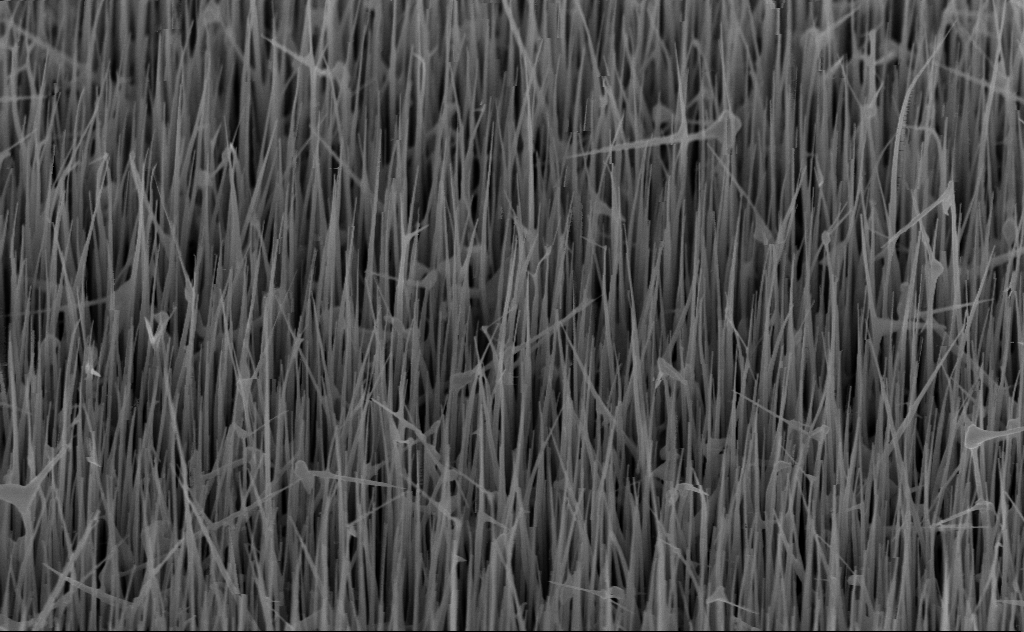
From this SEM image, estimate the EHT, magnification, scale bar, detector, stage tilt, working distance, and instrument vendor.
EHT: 10 kV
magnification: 40 K X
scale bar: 1000 nm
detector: InLens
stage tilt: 45°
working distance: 6 mm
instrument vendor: Zeiss